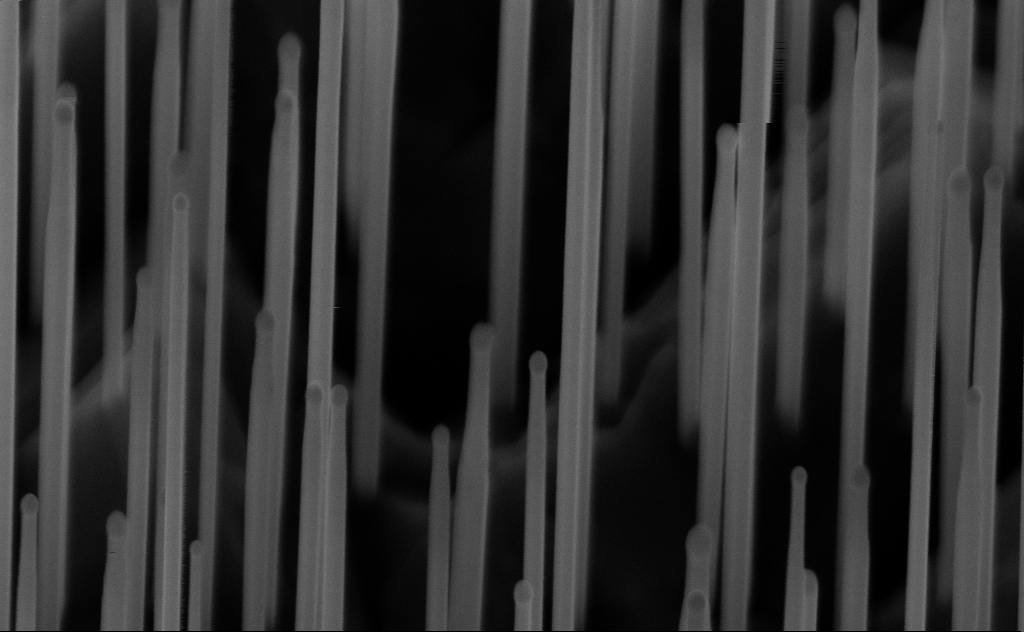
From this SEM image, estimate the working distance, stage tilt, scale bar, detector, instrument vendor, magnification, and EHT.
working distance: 6 mm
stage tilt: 45°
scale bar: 200 nm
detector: InLens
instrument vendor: Zeiss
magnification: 113.14 K X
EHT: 10 kV